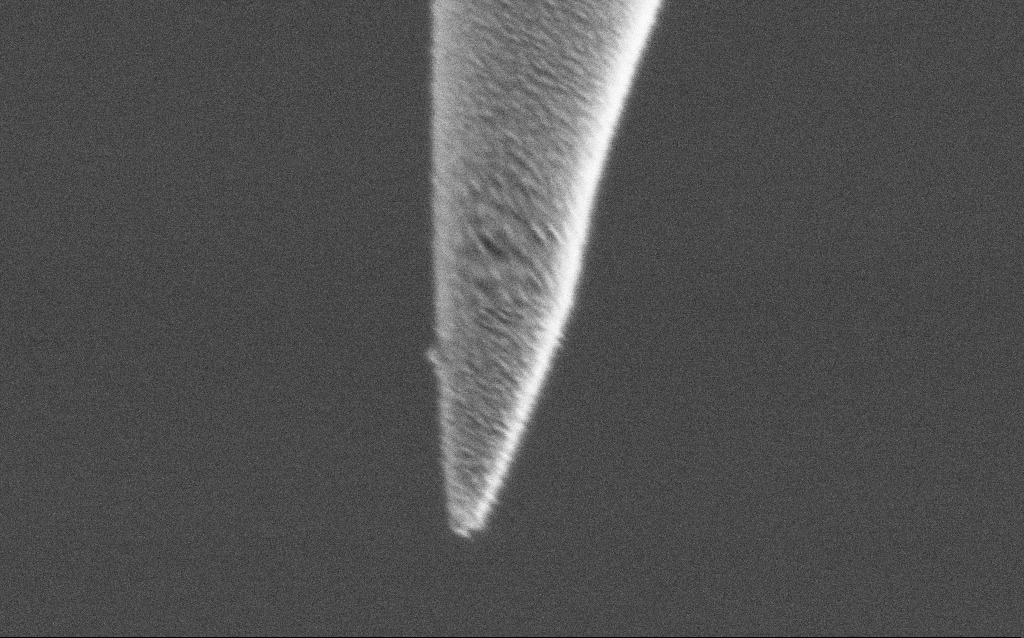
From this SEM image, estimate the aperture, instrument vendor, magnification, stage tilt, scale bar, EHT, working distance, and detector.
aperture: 30 µm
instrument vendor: Zeiss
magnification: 100 K X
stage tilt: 45°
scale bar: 200 nm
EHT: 1 kV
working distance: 6.9 mm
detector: SE2